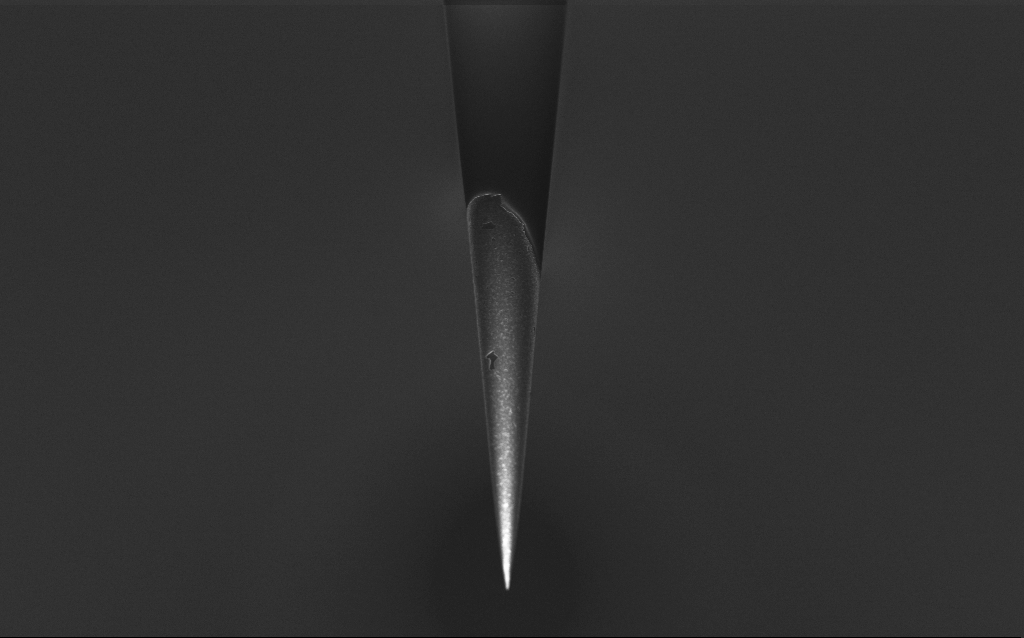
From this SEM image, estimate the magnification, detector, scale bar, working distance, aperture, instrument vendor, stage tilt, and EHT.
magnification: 5 K X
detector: InLens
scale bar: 10000 nm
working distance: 6 mm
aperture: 30 µm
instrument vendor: Zeiss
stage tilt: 45°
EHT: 1 kV